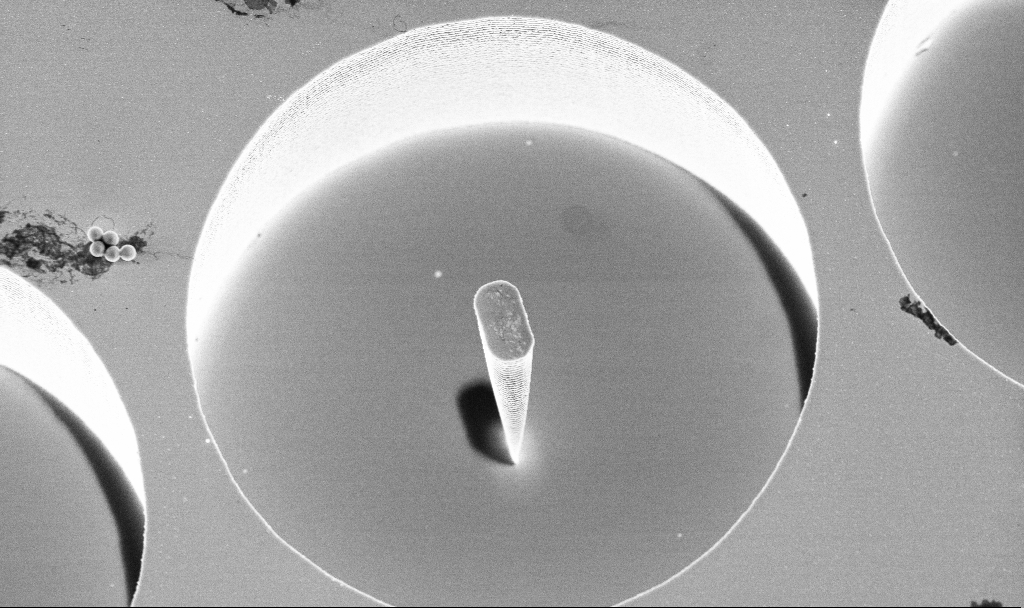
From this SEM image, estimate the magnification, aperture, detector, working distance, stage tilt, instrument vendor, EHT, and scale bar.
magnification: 7.83 K X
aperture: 30 µm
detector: InLens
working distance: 4.7 mm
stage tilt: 15°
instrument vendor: Zeiss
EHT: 4 kV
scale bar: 2000 nm